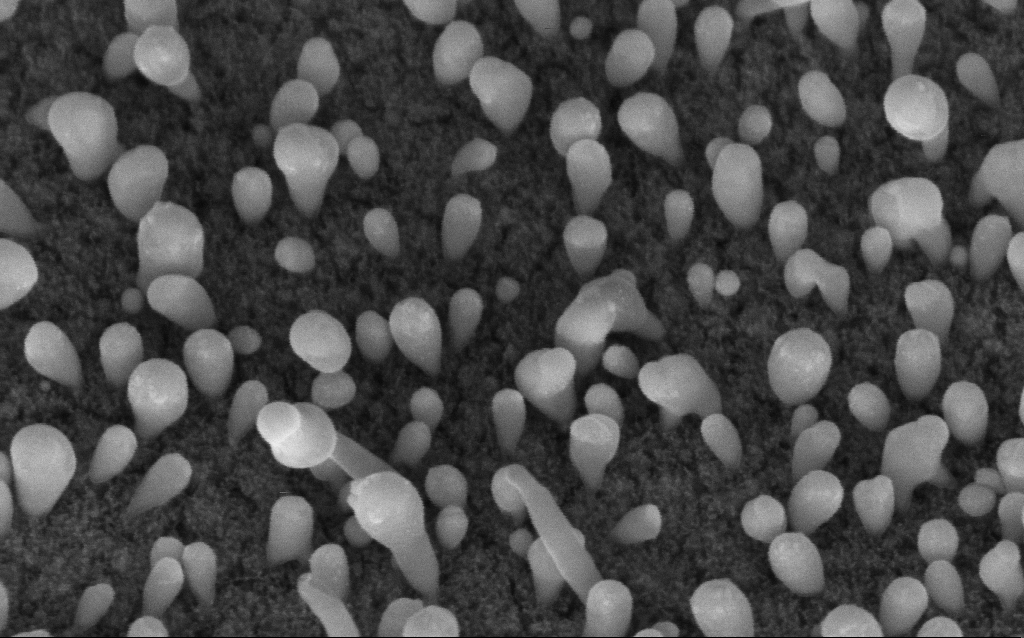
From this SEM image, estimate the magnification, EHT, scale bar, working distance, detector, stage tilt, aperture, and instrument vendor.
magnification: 200 K X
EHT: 5 kV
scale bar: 100 nm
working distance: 6.2 mm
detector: InLens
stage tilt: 45°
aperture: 30 µm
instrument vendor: Zeiss